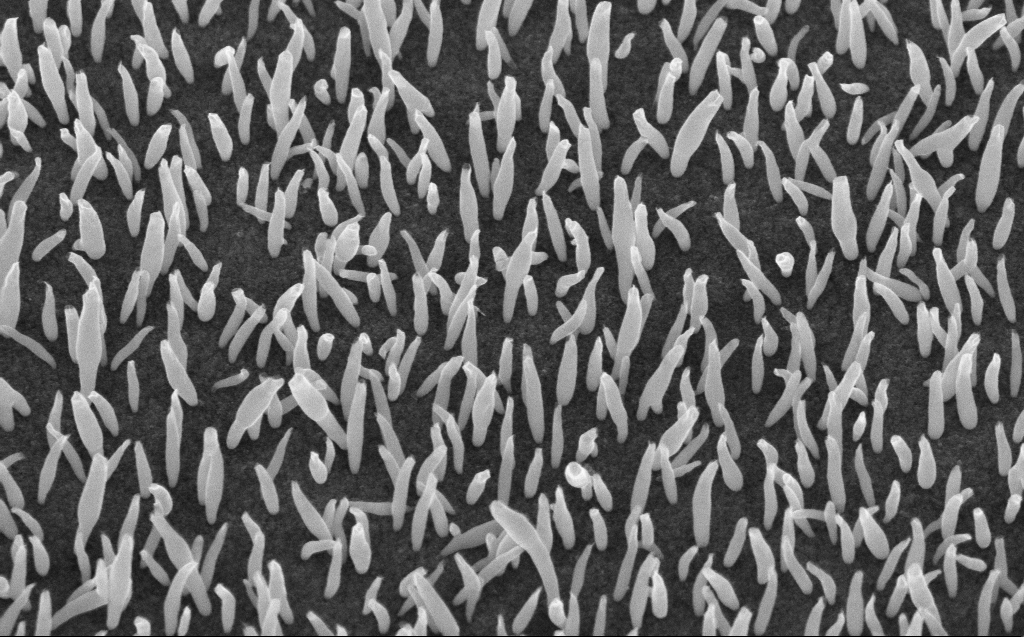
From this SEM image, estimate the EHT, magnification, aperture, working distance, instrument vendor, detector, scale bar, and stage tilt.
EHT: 10 kV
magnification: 50 K X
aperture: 30 µm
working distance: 6 mm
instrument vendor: Zeiss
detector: InLens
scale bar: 1000 nm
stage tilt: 45°